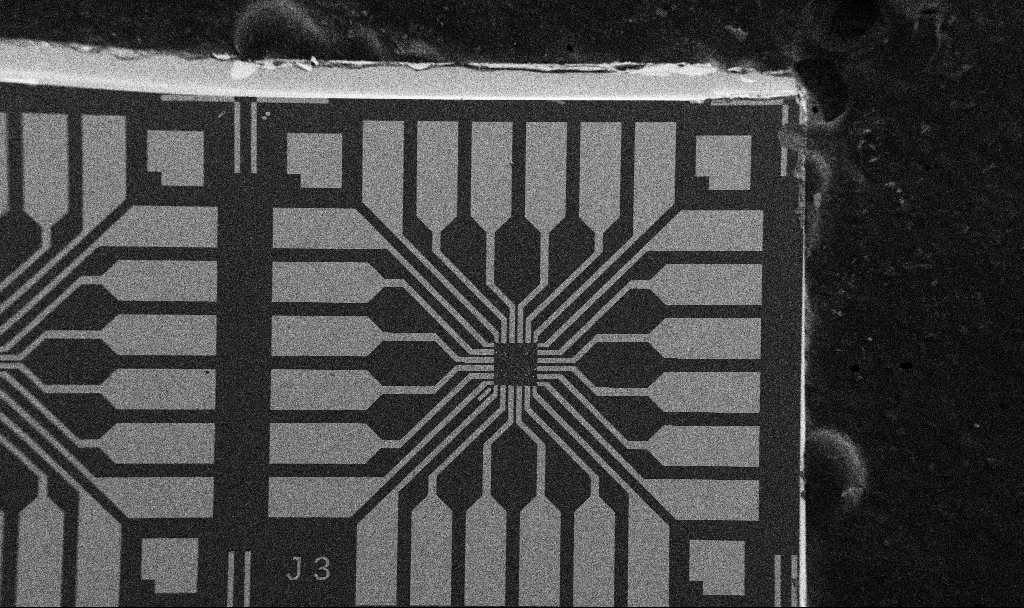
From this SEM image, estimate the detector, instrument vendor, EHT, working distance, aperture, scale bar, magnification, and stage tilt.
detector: SE2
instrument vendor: Zeiss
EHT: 5 kV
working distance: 10.7 mm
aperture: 30 µm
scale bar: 200000 nm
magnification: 0.1 K X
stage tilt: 0°